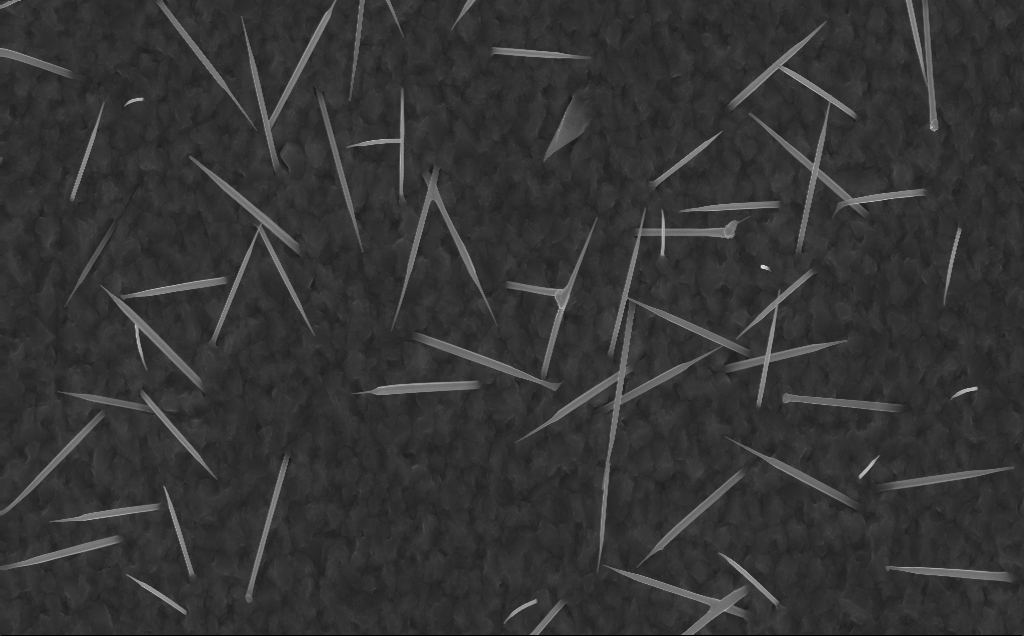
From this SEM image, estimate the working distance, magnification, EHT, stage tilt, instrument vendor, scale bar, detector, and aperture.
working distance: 5 mm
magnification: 20 K X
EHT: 10 kV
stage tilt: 0°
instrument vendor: Zeiss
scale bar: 2000 nm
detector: InLens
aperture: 30 µm